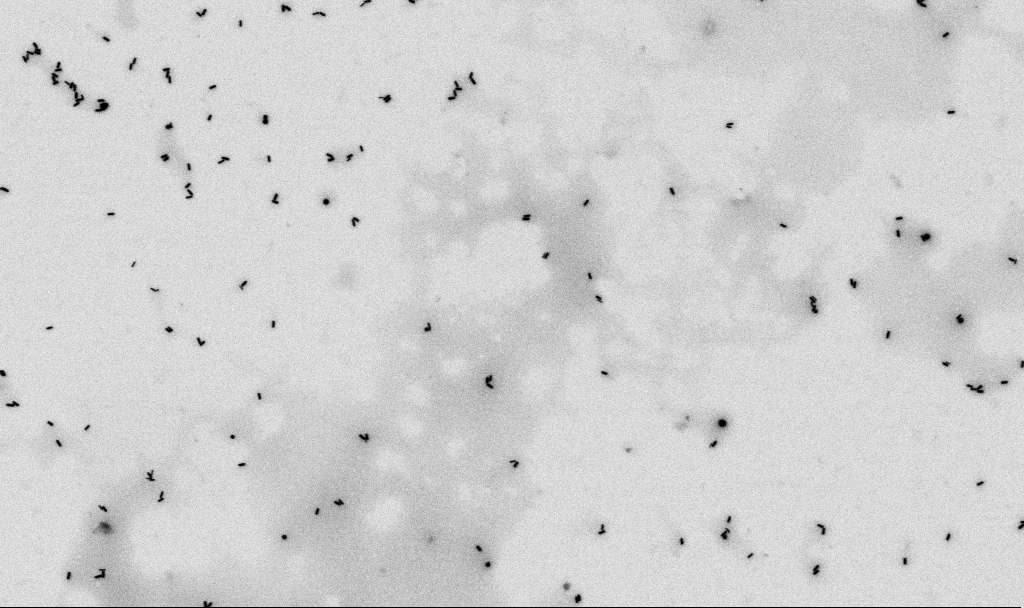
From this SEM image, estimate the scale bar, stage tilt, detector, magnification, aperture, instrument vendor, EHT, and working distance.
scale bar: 1000 nm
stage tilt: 0°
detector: SE2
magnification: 32.53 K X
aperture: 30 µm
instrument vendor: Zeiss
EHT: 2 kV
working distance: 4.5 mm